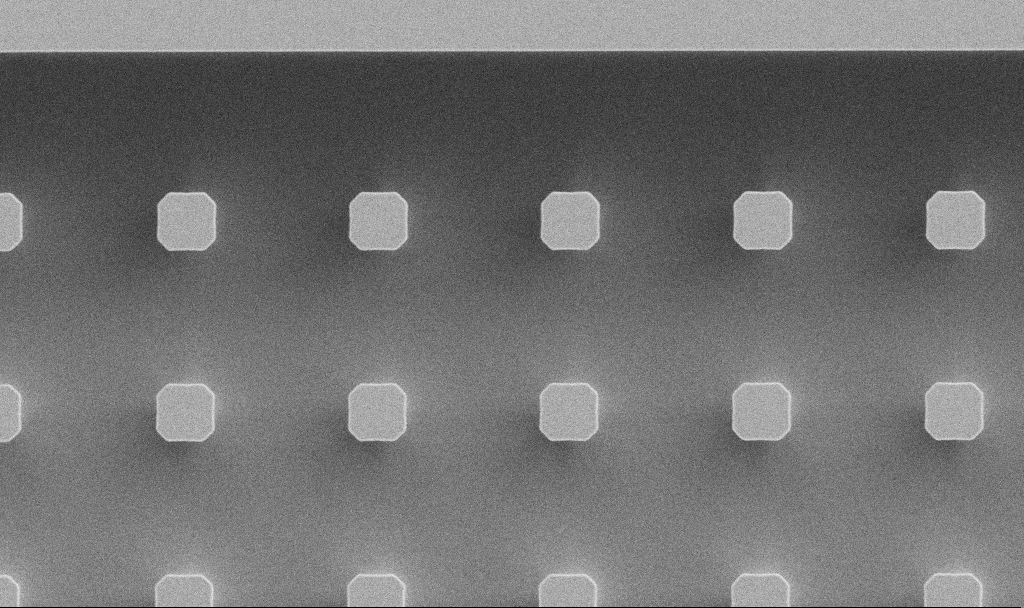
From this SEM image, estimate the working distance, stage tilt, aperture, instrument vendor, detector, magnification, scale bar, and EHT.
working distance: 7.7 mm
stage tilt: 0°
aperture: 30 µm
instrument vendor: Zeiss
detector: SE2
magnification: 2.36 K X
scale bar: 20000 nm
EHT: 5 kV